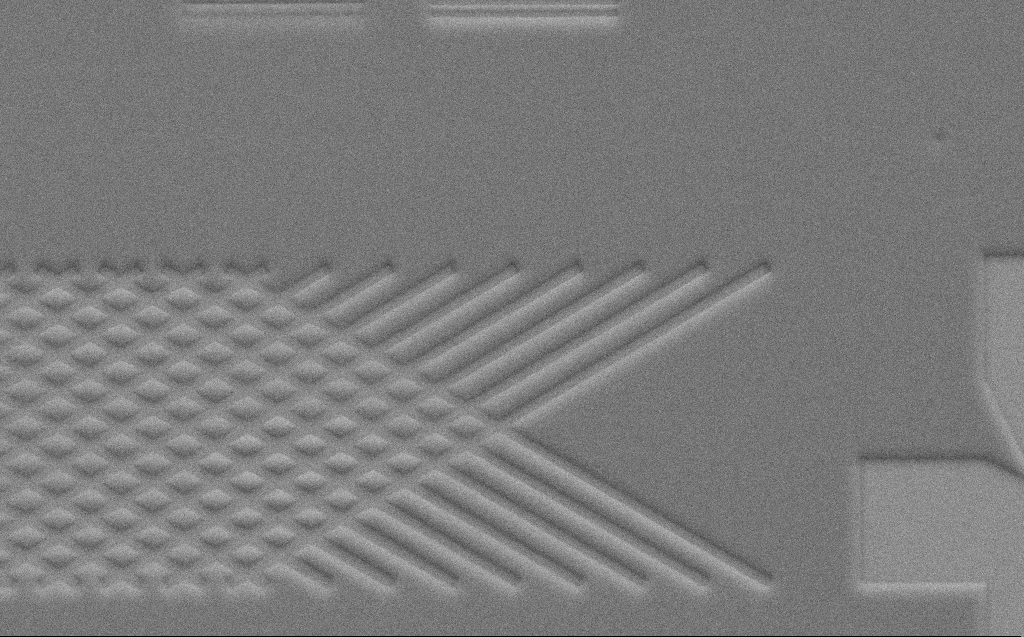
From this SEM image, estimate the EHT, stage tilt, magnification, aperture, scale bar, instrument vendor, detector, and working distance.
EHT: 10 kV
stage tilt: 45°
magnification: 4.63 K X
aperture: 30 µm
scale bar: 10000 nm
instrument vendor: Zeiss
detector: SE2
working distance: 6 mm